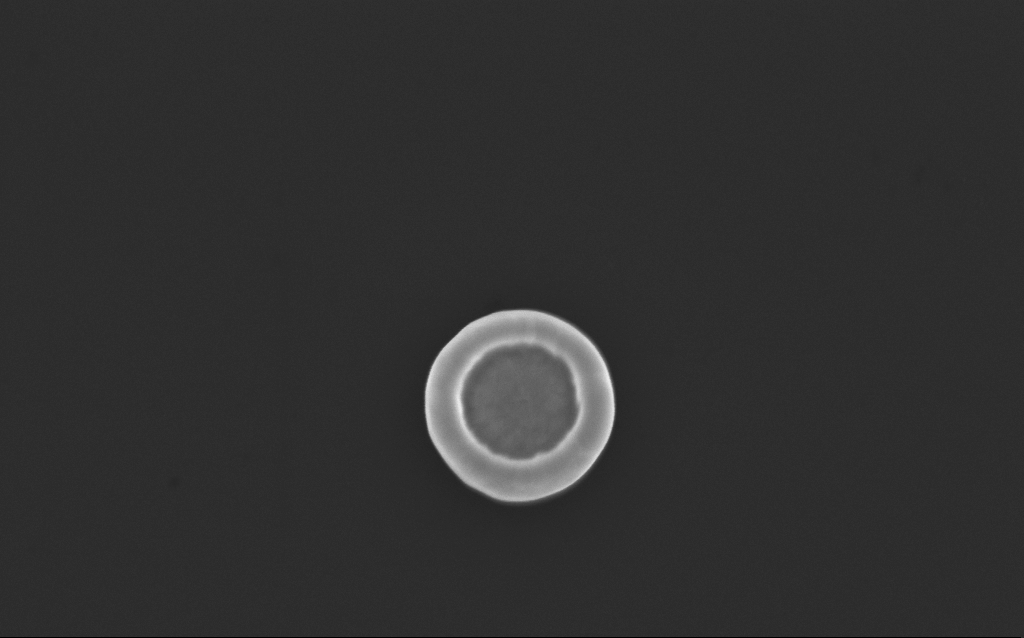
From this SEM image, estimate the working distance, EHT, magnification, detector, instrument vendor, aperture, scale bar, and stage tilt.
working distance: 4 mm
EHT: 10 kV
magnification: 55.2 K X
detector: InLens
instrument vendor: Zeiss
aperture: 30 µm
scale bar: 1000 nm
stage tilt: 0°